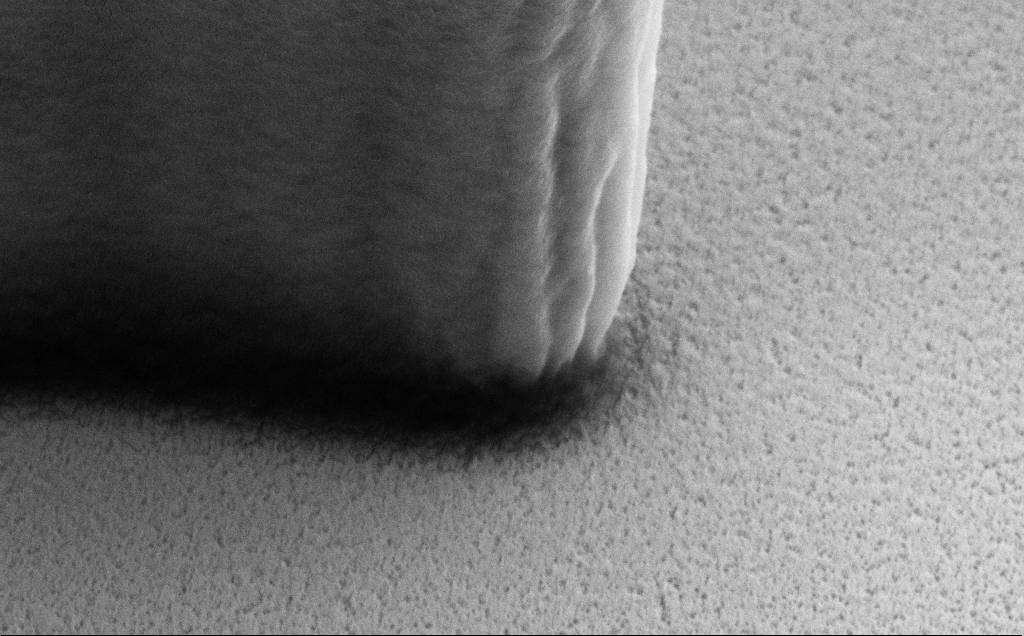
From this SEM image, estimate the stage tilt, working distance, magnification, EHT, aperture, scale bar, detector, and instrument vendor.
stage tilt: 40°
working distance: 9 mm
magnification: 74.49 K X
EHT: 5 kV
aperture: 30 µm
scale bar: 200 nm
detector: SE2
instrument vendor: Zeiss